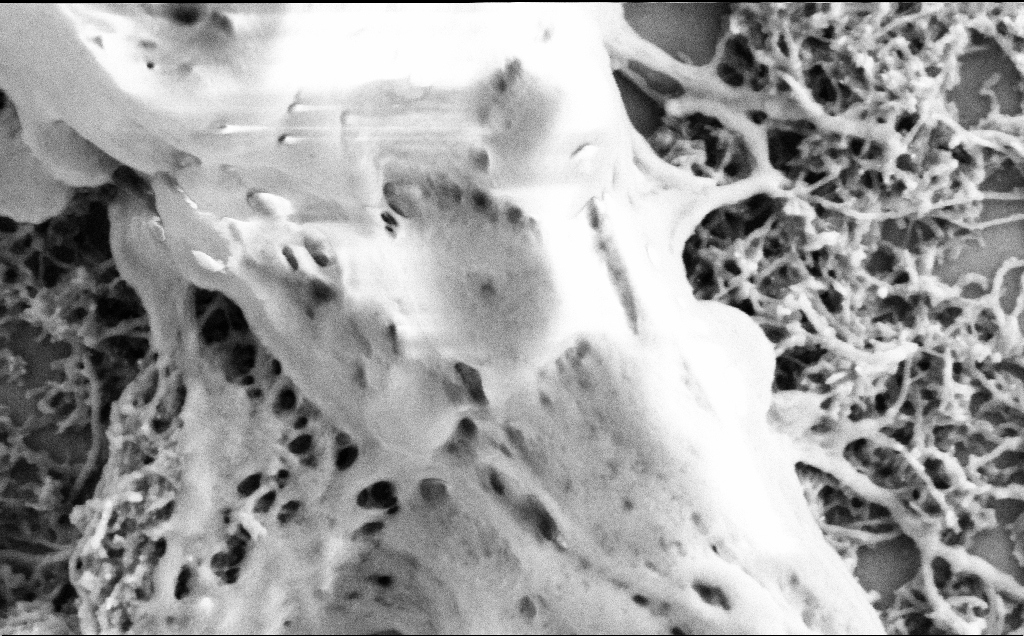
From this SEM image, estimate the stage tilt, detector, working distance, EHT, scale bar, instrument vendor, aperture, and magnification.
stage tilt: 0°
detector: SE2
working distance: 7.1 mm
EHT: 2 kV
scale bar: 200 nm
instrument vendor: Zeiss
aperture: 30 µm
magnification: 75 K X